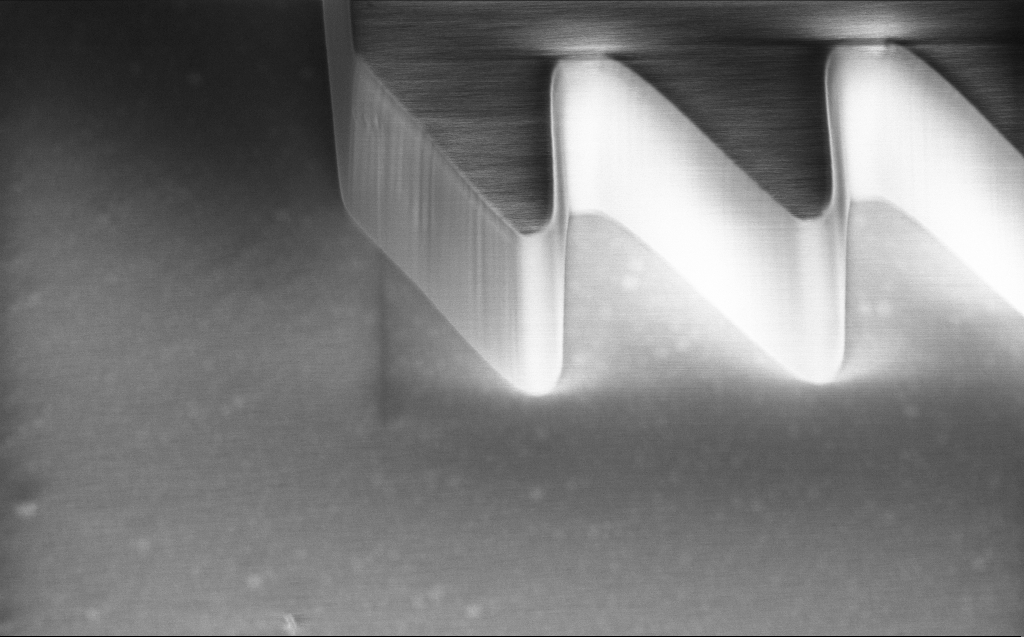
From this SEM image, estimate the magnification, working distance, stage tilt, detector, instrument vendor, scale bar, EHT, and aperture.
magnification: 8.95 K X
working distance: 7 mm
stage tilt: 30°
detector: InLens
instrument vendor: Zeiss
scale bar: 2000 nm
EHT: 1 kV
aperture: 30 µm